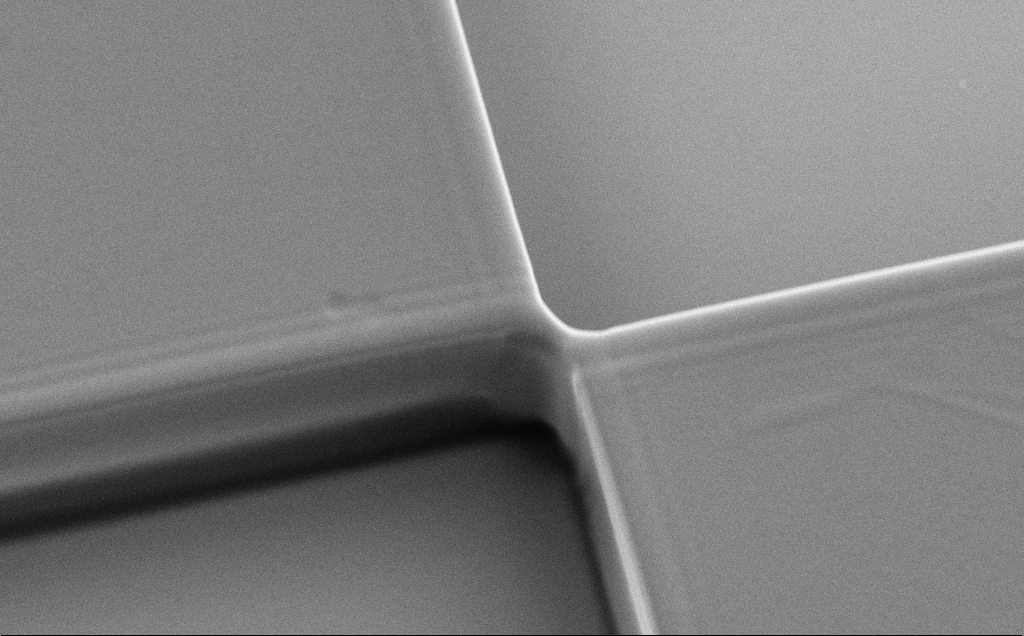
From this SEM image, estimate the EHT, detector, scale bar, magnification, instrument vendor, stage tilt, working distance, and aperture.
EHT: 5 kV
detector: SE2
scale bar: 2000 nm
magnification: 7.67 K X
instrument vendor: Zeiss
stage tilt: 30°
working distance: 11 mm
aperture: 30 µm